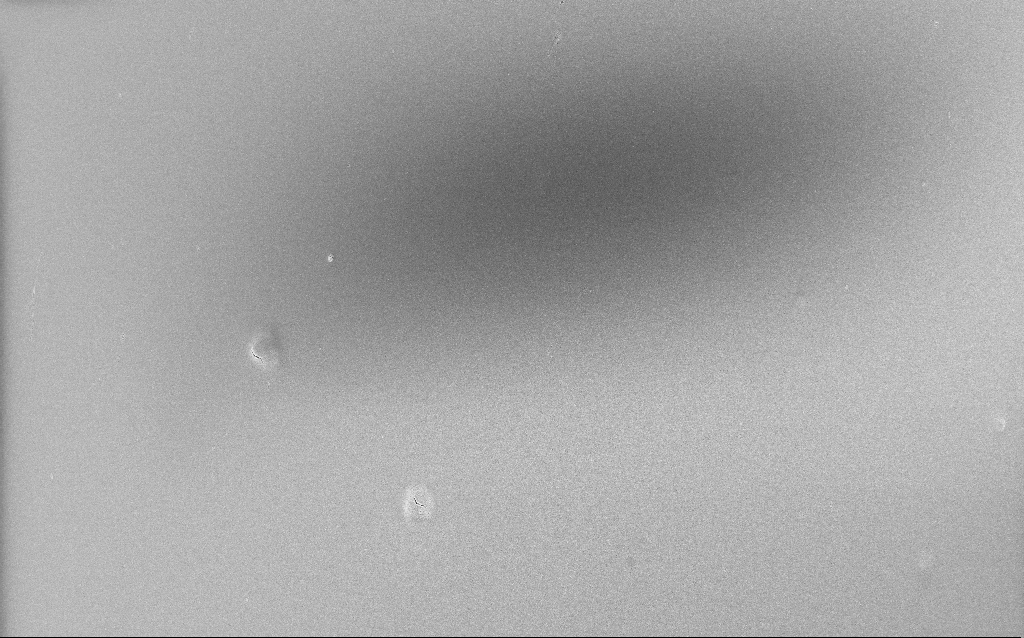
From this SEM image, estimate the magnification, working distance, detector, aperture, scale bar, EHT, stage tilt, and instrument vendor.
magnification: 0.792 K X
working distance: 2.6 mm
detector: InLens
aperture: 30 µm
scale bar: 20000 nm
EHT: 5 kV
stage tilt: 0°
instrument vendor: Zeiss